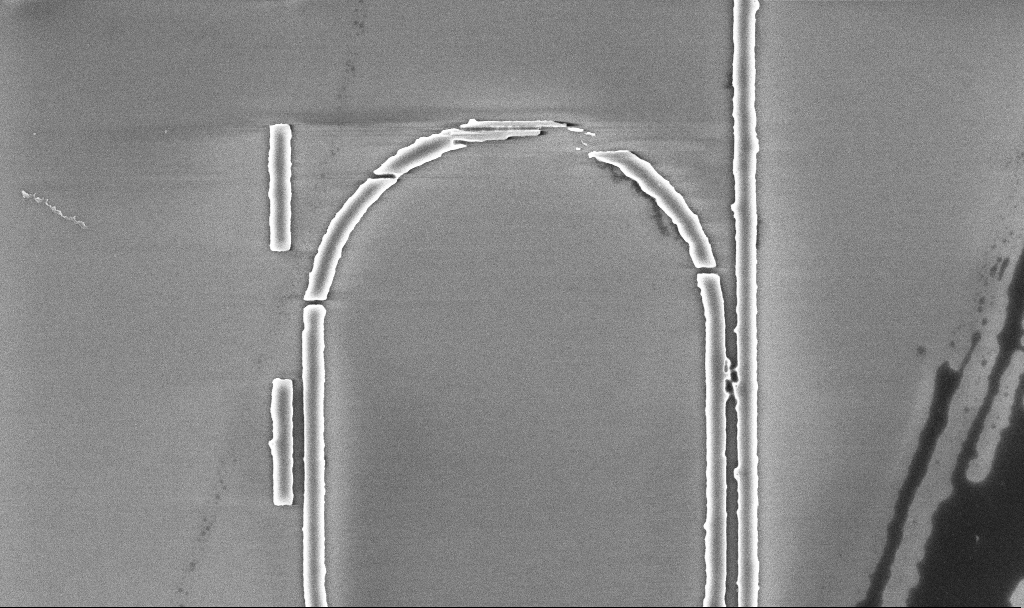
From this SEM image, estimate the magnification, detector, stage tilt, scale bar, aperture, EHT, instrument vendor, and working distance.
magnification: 15.72 K X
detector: InLens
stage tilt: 0°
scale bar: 2000 nm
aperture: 30 µm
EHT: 5 kV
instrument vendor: Zeiss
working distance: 5.2 mm